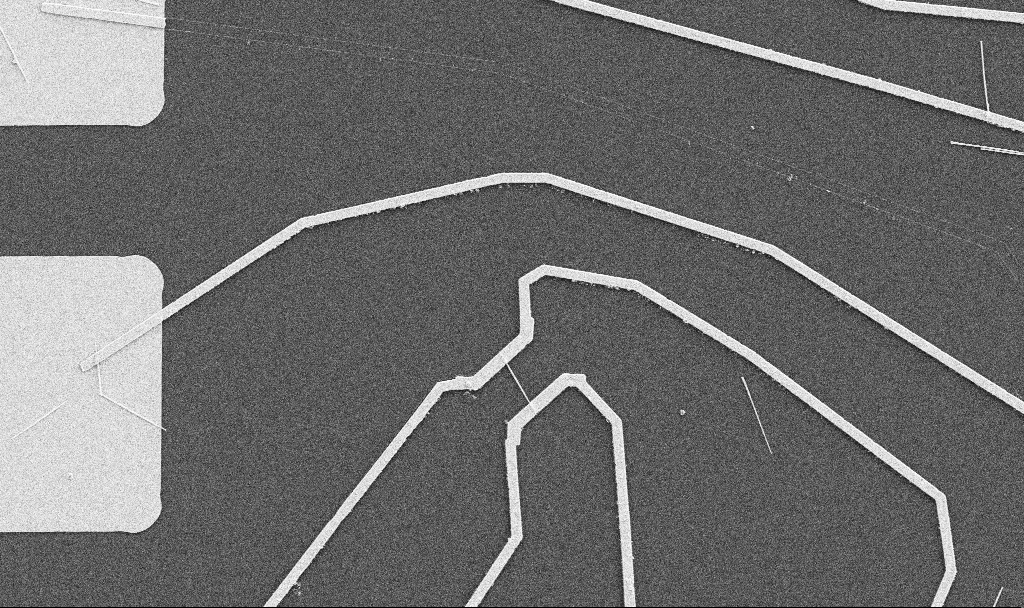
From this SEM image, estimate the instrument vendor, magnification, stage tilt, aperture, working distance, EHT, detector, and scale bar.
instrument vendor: Zeiss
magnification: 5 K X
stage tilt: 0°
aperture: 30 µm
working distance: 10.7 mm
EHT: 5 kV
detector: SE2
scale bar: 10000 nm